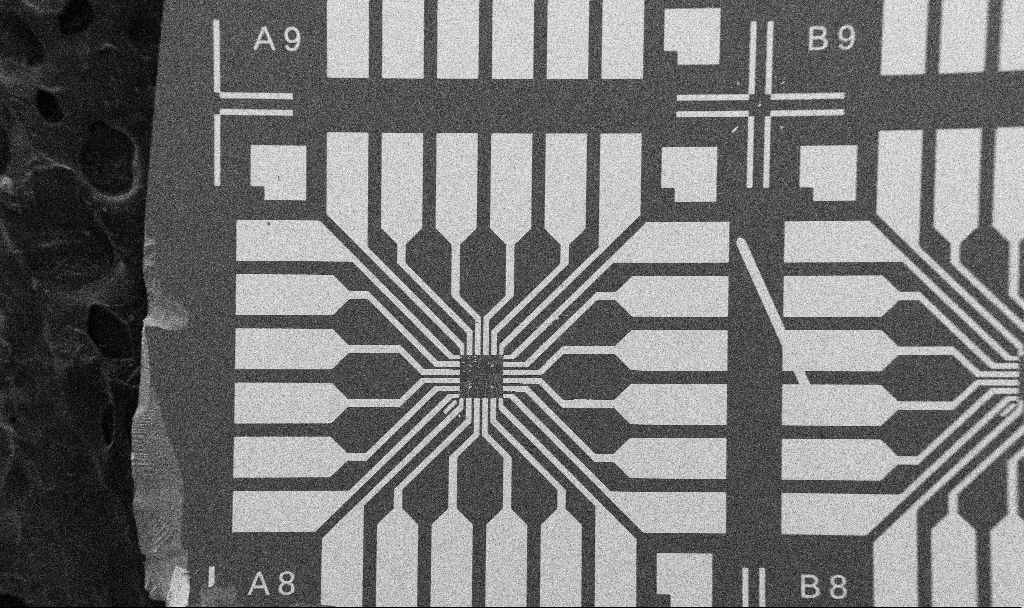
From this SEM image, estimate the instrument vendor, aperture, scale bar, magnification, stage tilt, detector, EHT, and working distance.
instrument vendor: Zeiss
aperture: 30 µm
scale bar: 200000 nm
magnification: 0.1 K X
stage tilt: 0°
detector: SE2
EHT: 5 kV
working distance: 10.7 mm